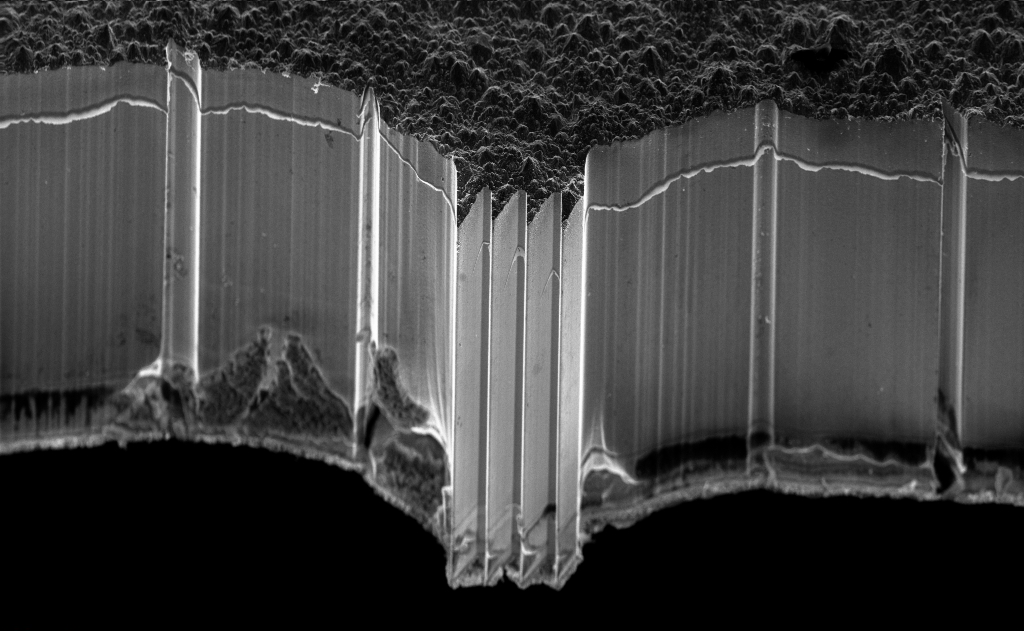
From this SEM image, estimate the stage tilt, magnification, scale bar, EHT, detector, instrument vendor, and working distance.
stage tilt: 45°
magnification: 1.12 K X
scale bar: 20000 nm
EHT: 10 kV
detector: InLens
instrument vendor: Zeiss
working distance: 4 mm